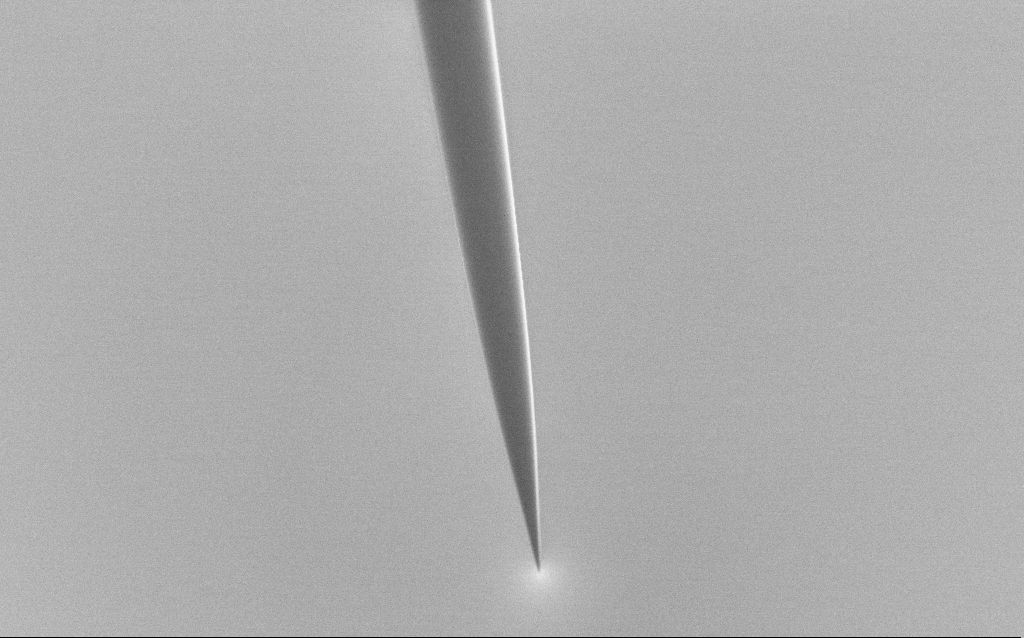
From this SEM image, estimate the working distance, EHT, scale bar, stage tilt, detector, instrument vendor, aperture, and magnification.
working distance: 6 mm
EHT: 1 kV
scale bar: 20000 nm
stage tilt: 45°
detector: SE2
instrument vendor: Zeiss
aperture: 30 µm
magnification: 1 K X